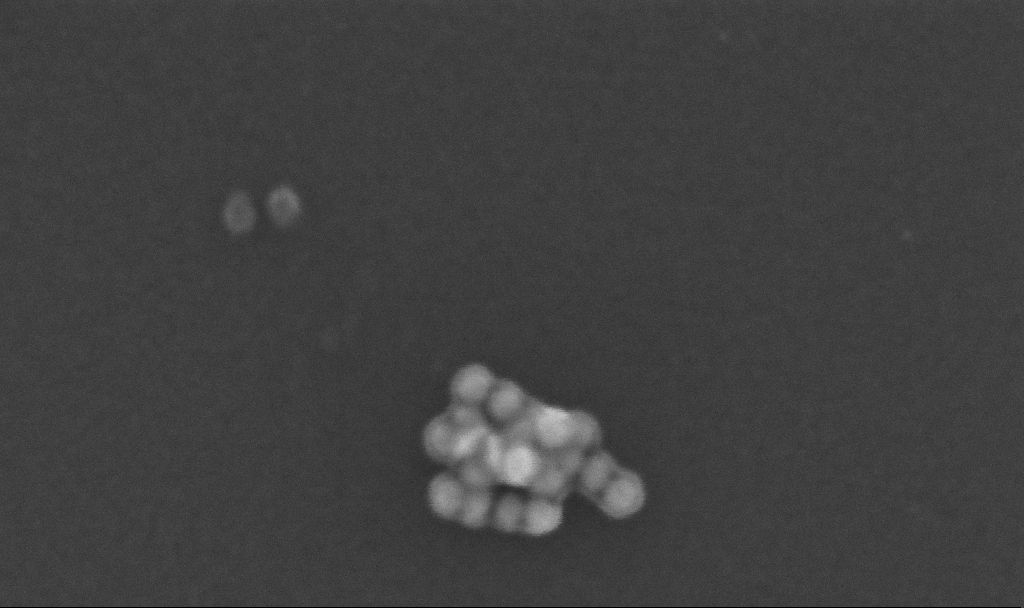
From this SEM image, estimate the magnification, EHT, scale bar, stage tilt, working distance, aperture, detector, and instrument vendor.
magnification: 682.51 K X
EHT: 10 kV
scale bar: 100 nm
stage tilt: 0°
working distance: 3.2 mm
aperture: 30 µm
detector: InLens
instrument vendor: Zeiss